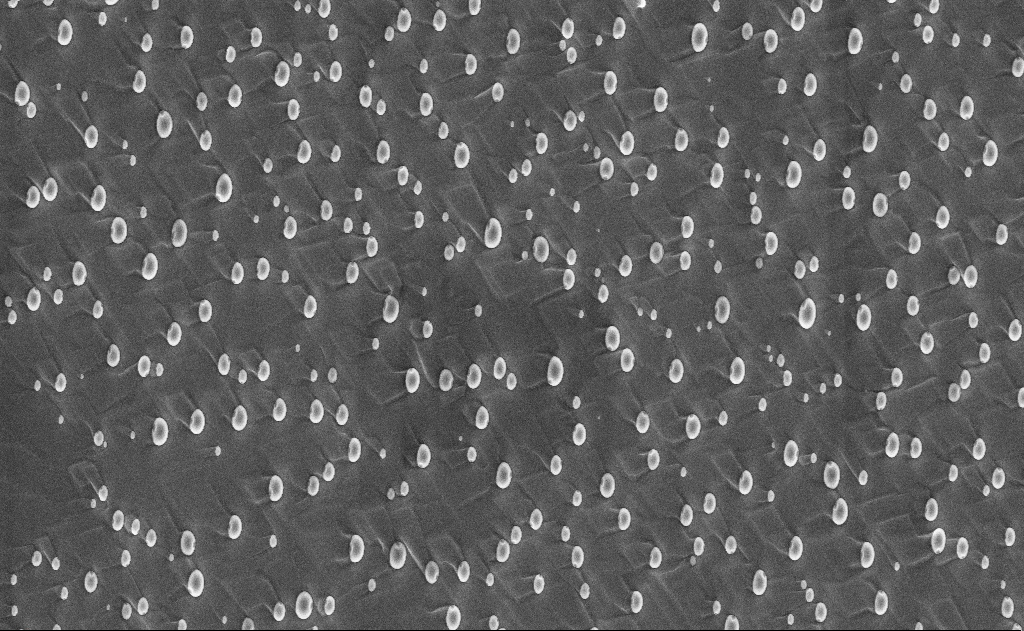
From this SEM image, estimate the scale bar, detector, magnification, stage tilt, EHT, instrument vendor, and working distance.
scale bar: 10000 nm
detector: InLens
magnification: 5 K X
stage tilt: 0°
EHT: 10 kV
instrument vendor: Zeiss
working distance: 15 mm